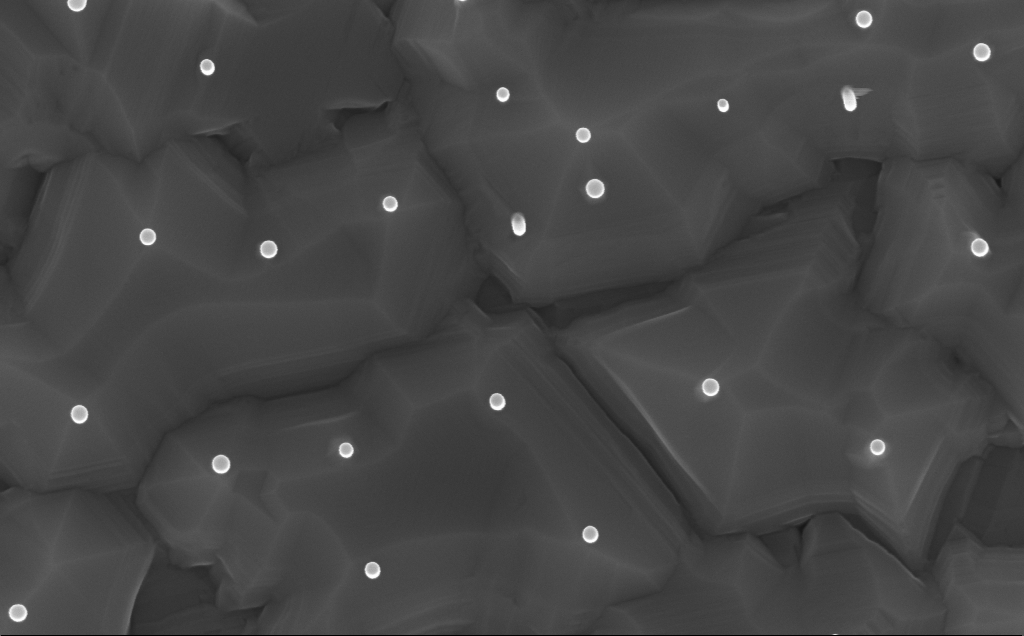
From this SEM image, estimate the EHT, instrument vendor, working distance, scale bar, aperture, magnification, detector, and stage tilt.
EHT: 10 kV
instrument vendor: Zeiss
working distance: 7 mm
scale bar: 200 nm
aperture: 30 µm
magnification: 80 K X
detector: InLens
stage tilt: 0.2°